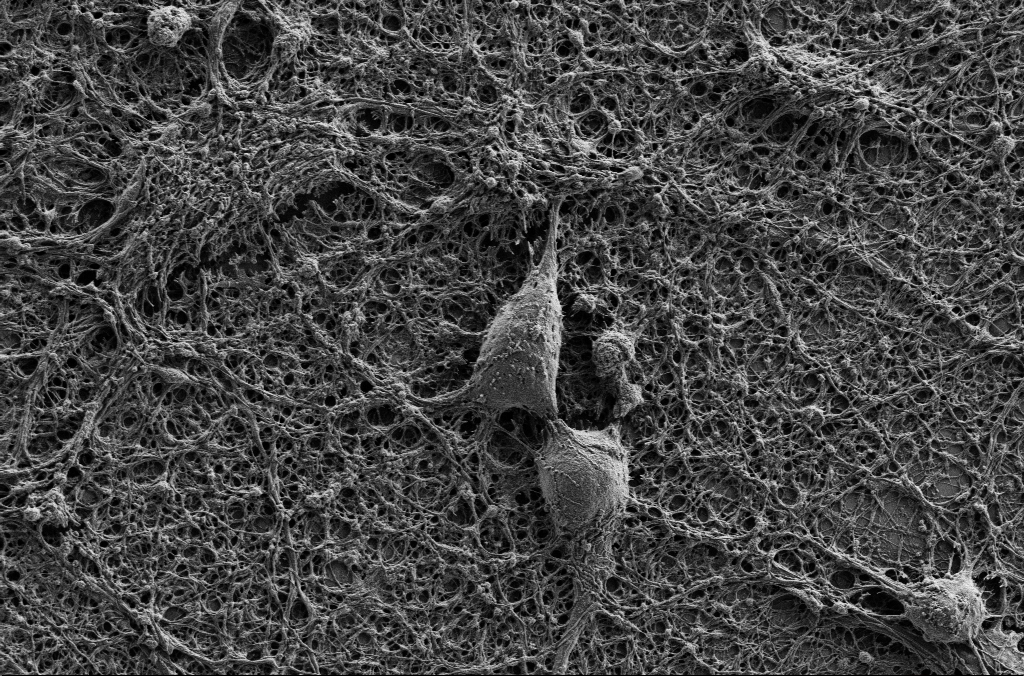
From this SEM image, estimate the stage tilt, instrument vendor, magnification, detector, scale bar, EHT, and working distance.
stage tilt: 0°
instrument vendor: Zeiss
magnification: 5 K X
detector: SE2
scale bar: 10000 nm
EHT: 1 kV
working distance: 4.1 mm